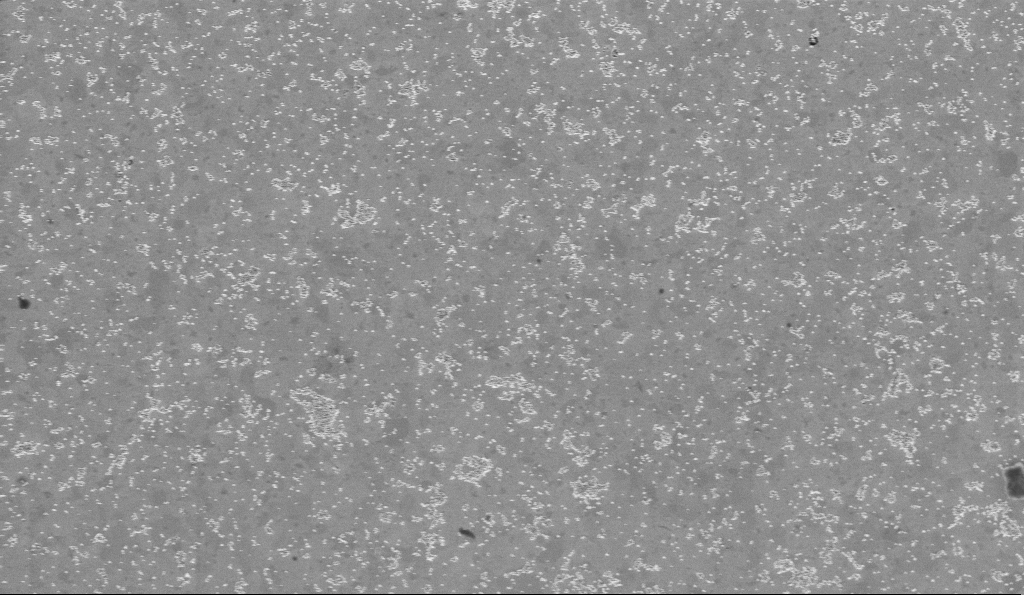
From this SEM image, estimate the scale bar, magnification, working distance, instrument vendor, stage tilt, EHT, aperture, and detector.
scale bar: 1000 nm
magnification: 25 K X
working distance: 3.2 mm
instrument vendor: Zeiss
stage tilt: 0°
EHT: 2 kV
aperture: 30 µm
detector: InLens